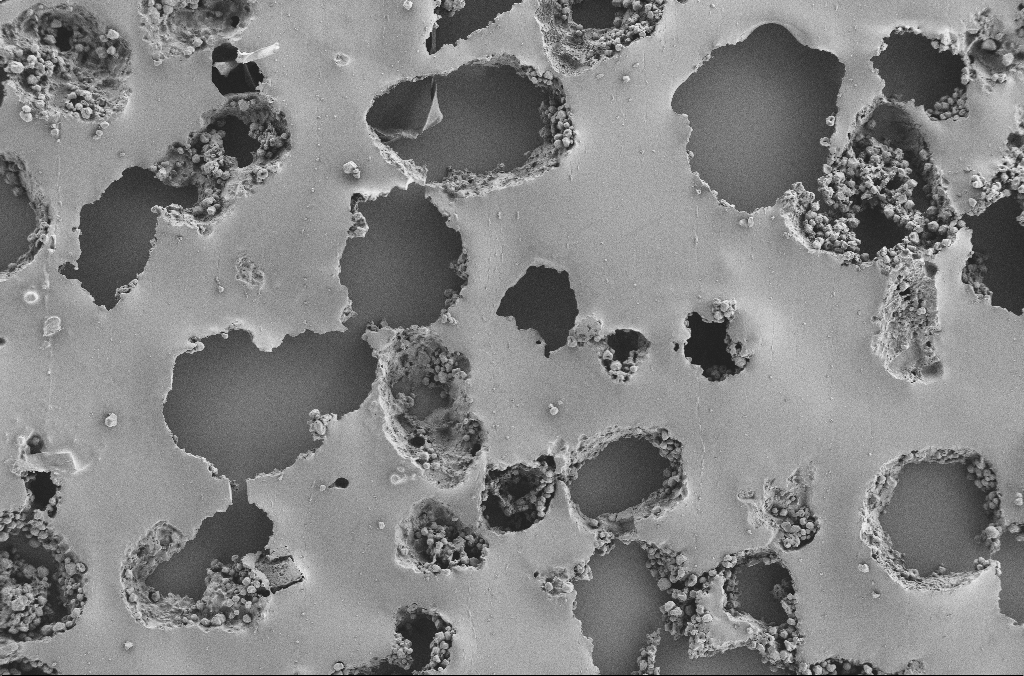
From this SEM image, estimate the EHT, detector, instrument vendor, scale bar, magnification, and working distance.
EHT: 2 kV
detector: SE2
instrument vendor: Zeiss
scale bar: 100000 nm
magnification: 0.25 K X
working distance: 3.7 mm